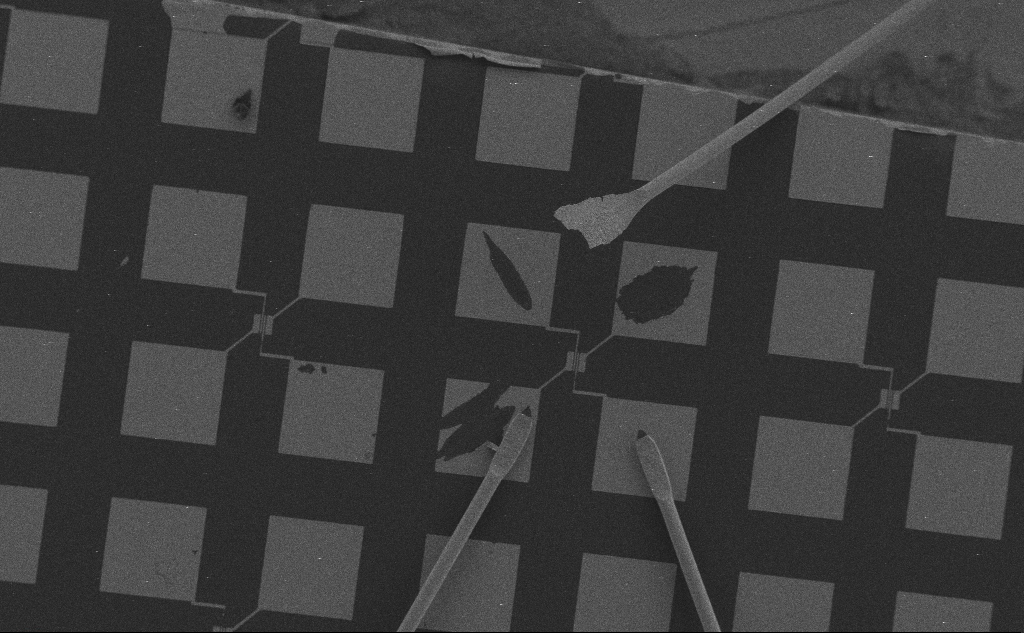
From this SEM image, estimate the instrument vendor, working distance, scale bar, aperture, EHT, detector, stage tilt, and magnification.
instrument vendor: Zeiss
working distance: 6 mm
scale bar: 100000 nm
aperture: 30 µm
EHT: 5 kV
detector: SE2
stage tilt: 0°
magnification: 0.237 K X